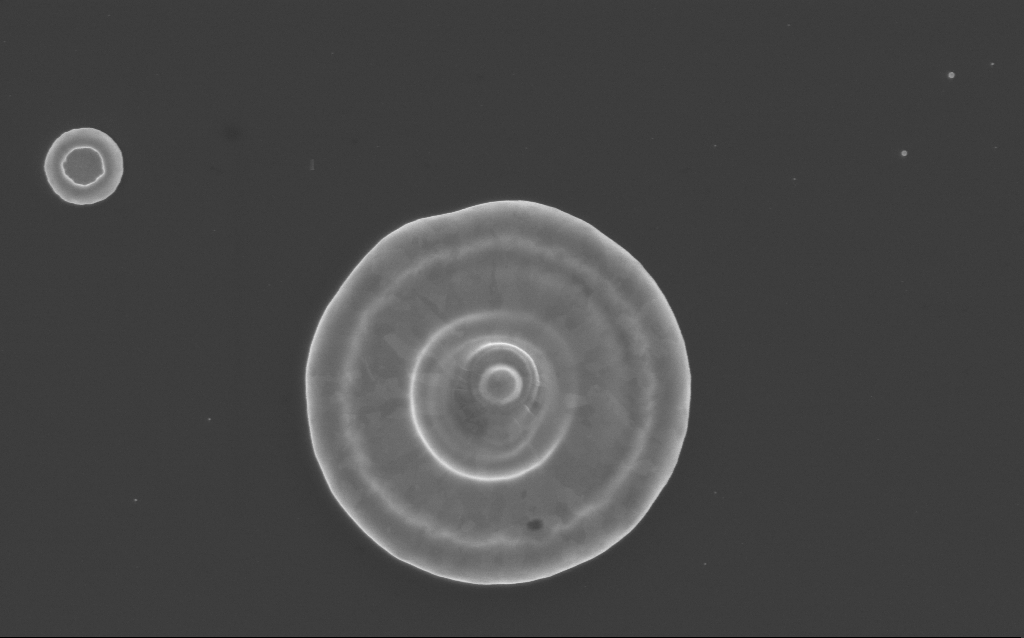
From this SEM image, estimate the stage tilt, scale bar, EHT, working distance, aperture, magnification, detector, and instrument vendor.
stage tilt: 0°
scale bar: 2000 nm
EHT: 3 kV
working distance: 3 mm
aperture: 30 µm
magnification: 30.26 K X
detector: InLens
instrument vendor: Zeiss